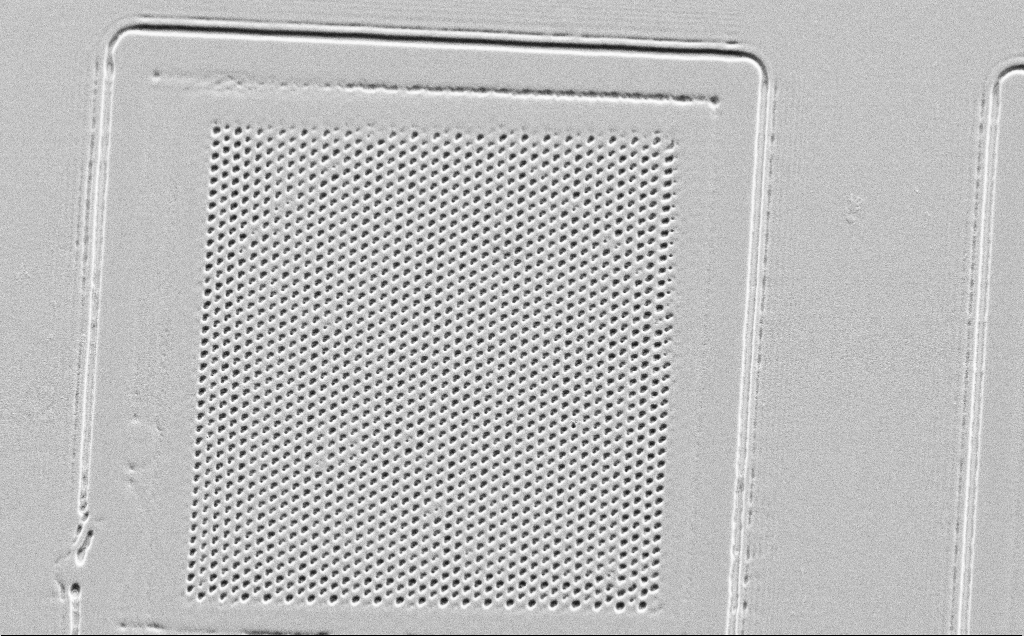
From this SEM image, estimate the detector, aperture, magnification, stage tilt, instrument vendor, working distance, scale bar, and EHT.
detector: SE2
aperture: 30 µm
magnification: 2.32 K X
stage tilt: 45°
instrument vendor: Zeiss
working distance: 10 mm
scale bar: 10000 nm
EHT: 5 kV